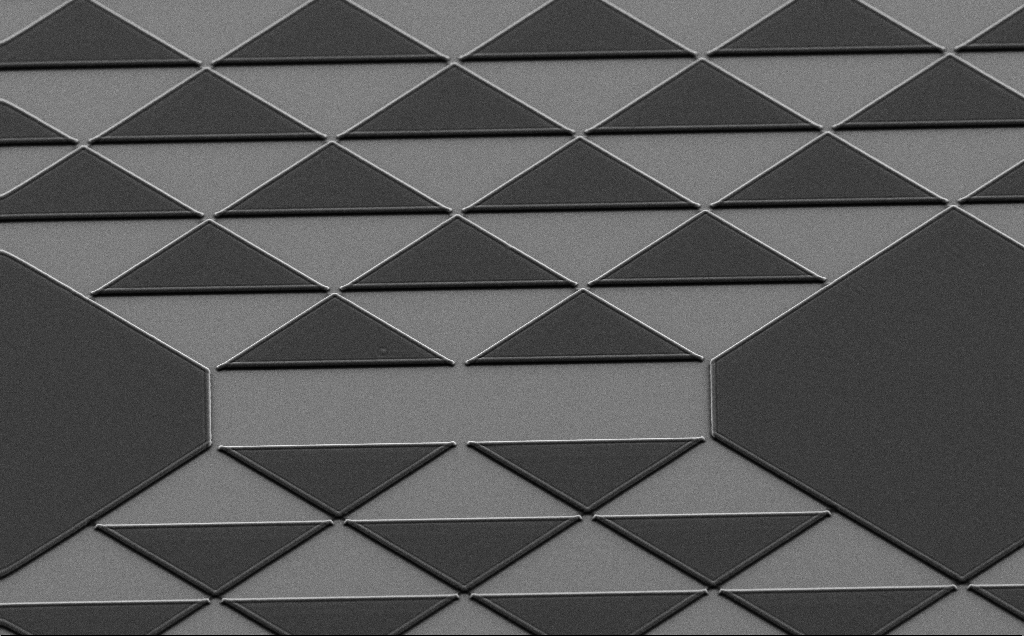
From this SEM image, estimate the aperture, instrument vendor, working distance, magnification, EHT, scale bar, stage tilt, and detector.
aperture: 30 µm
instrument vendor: Zeiss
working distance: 5 mm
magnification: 0.978 K X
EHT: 7 kV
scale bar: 20000 nm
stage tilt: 35°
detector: SE2